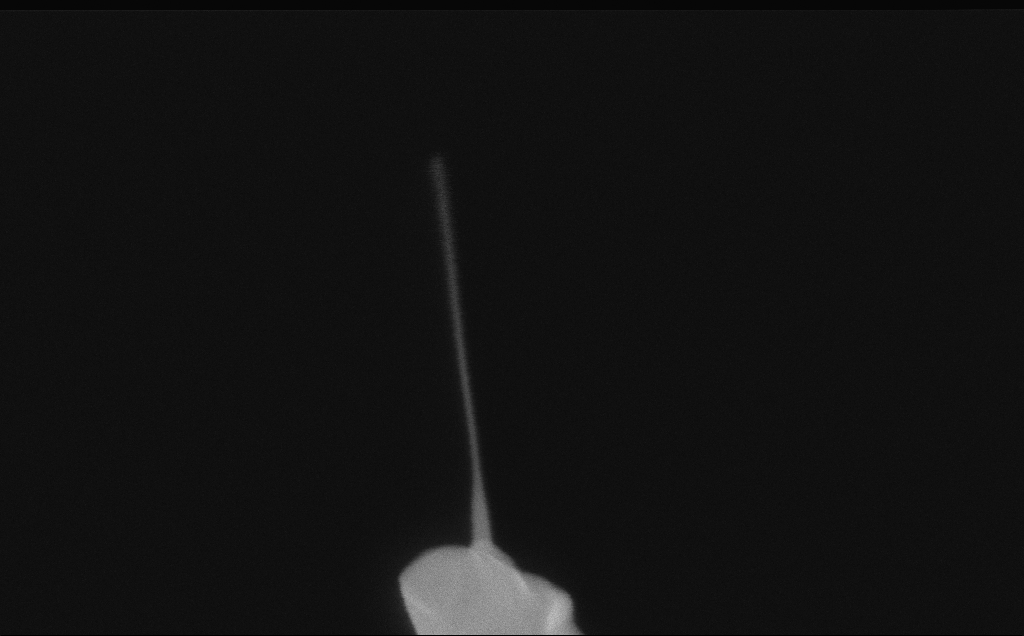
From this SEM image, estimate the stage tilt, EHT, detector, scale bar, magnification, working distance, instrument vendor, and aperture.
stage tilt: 0°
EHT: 10 kV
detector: InLens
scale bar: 200 nm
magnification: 257.27 K X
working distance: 6 mm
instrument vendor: Zeiss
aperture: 30 µm